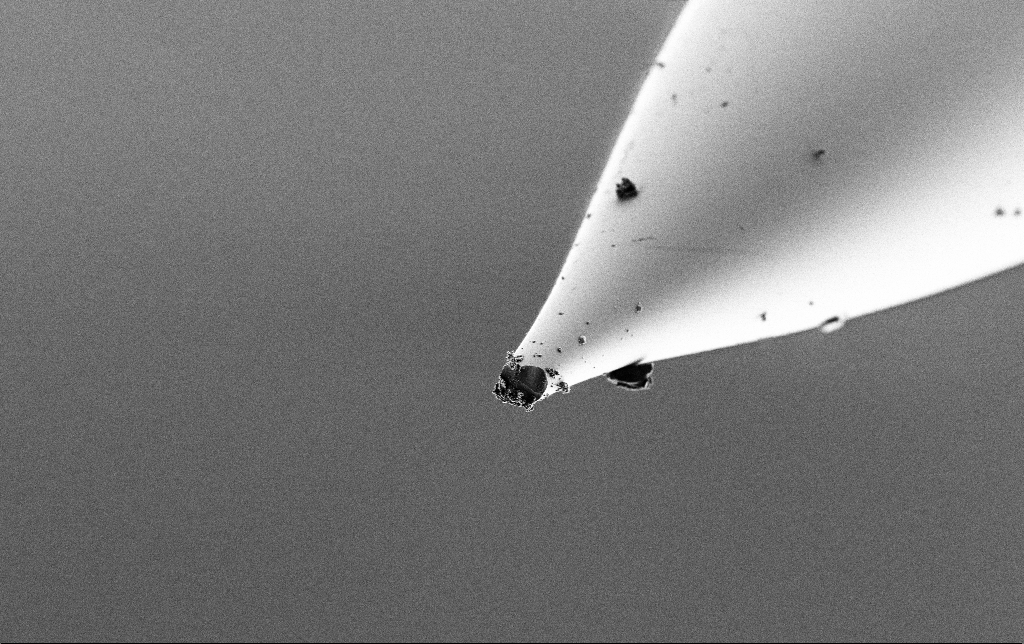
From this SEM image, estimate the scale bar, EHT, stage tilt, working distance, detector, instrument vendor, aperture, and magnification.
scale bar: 10000 nm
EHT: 2 kV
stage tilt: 70°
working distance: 6.2 mm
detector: SE2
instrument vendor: Zeiss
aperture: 30 µm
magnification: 2.15 K X